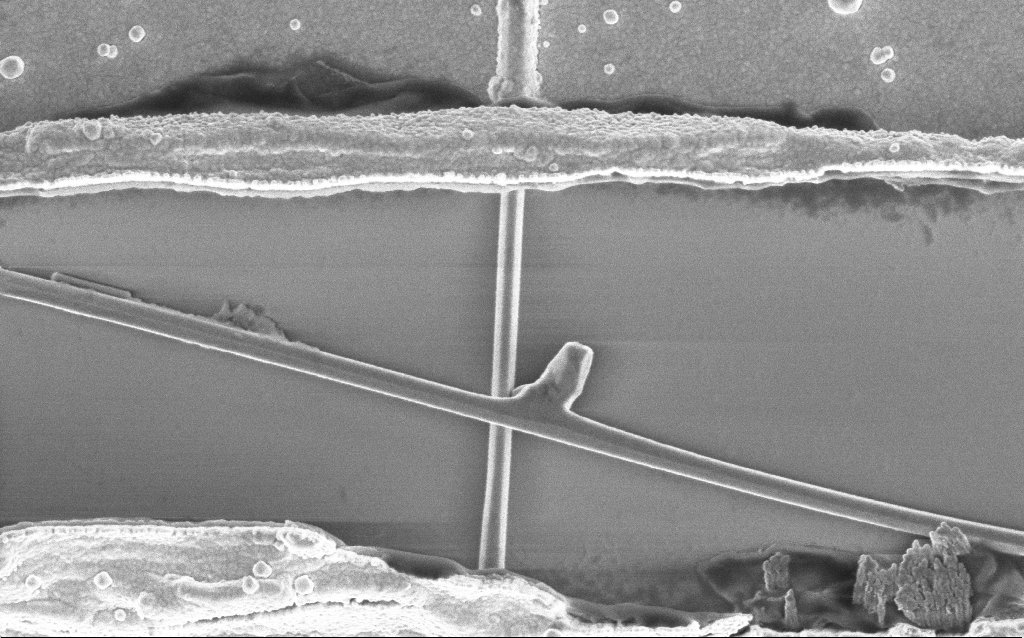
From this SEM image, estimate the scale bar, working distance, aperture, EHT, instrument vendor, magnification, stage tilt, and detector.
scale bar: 1000 nm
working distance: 7.7 mm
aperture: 30 µm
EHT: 2 kV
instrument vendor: Zeiss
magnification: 49.88 K X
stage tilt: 0°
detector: InLens